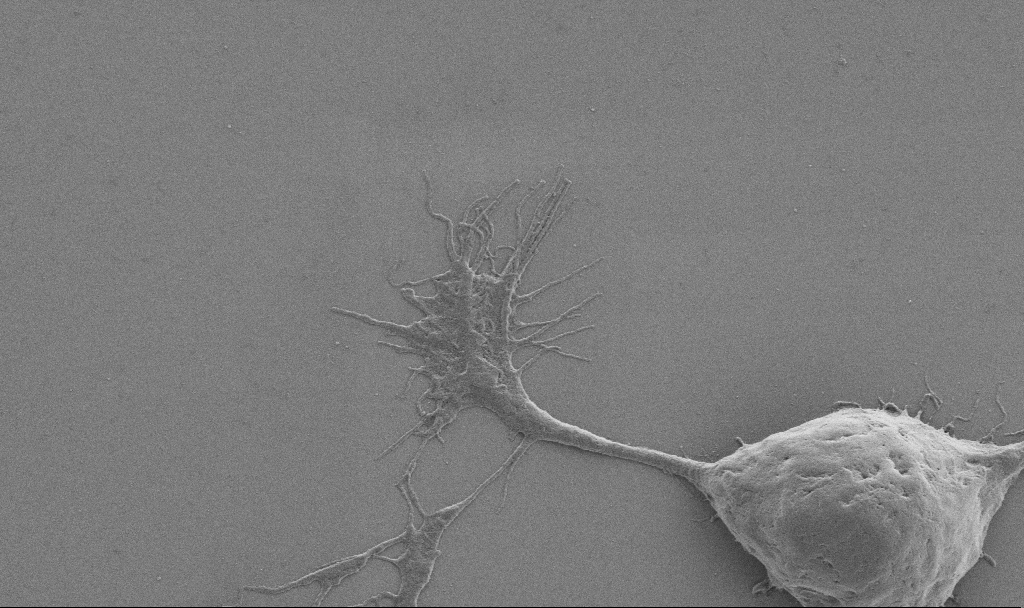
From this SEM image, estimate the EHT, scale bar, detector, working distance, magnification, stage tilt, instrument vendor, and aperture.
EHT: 0.9 kV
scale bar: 2000 nm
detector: SE2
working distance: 6.9 mm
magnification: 10 K X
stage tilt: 0°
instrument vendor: Zeiss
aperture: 30 µm